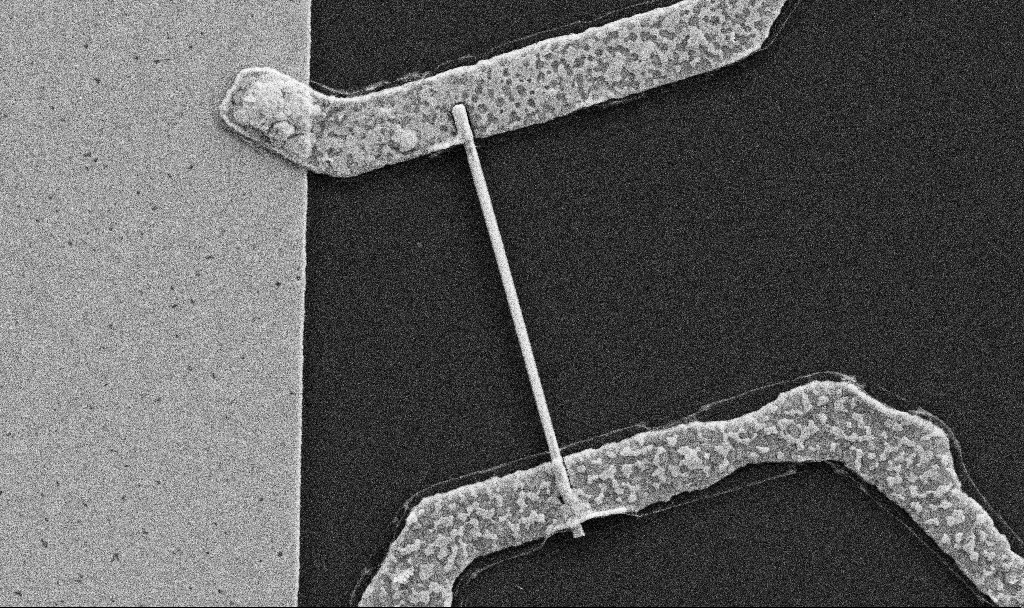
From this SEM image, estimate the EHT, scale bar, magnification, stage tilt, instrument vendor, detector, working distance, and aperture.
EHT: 5 kV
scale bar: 2000 nm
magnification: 30 K X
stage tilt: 0°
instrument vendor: Zeiss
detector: SE2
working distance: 6.7 mm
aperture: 30 µm